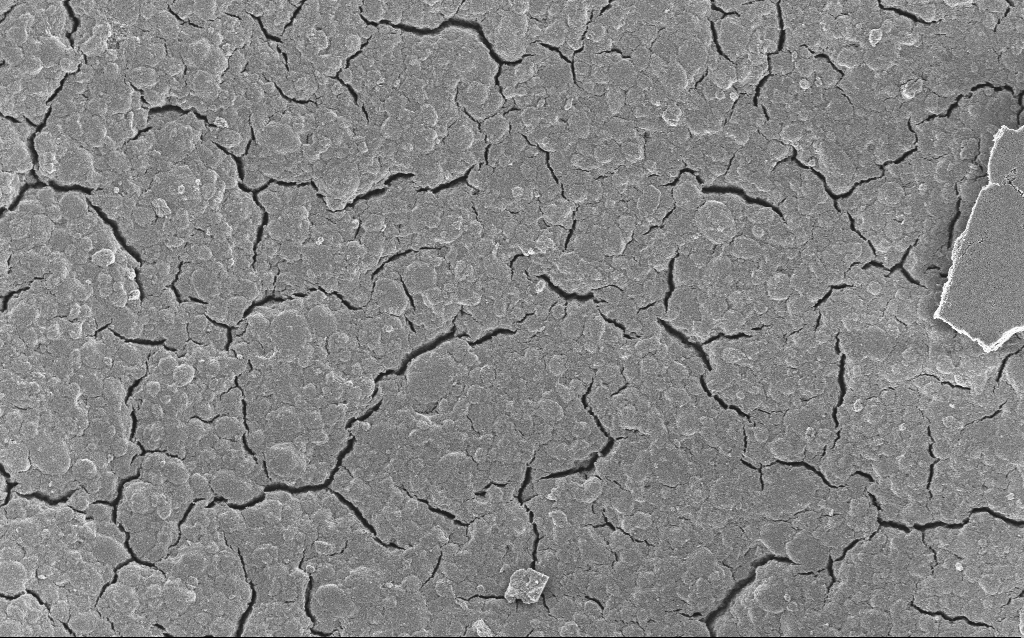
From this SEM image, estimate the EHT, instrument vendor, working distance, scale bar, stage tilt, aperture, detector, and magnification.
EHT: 5 kV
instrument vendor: Zeiss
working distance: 4.2 mm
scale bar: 10000 nm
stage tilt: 0°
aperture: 30 µm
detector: InLens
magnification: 1.36 K X